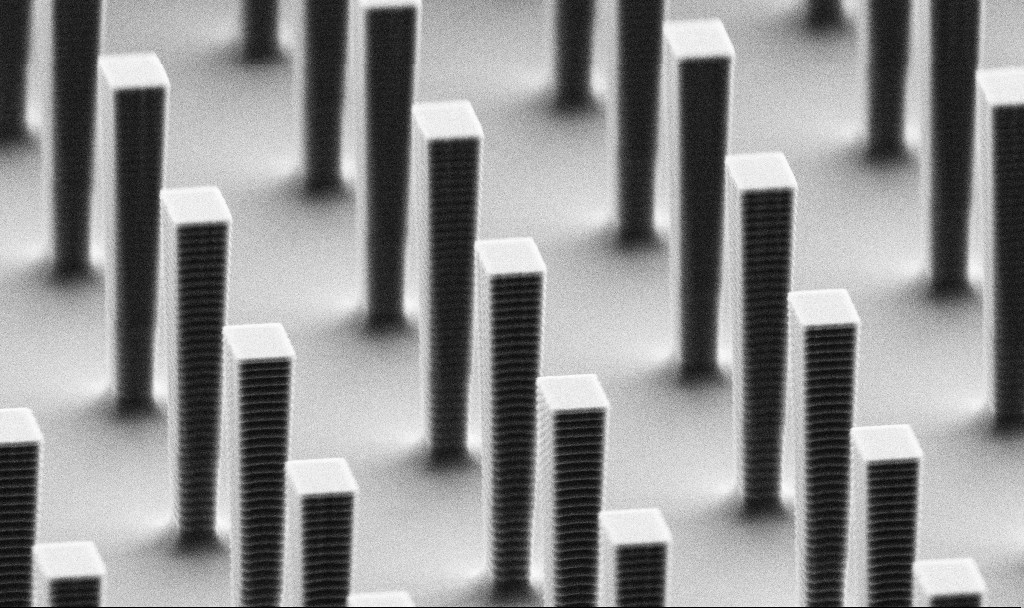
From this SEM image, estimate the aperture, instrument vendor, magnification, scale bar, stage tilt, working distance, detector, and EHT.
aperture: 30 µm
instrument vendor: Zeiss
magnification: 11.63 K X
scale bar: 2000 nm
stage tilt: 70°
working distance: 6.1 mm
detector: SE2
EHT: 5 kV